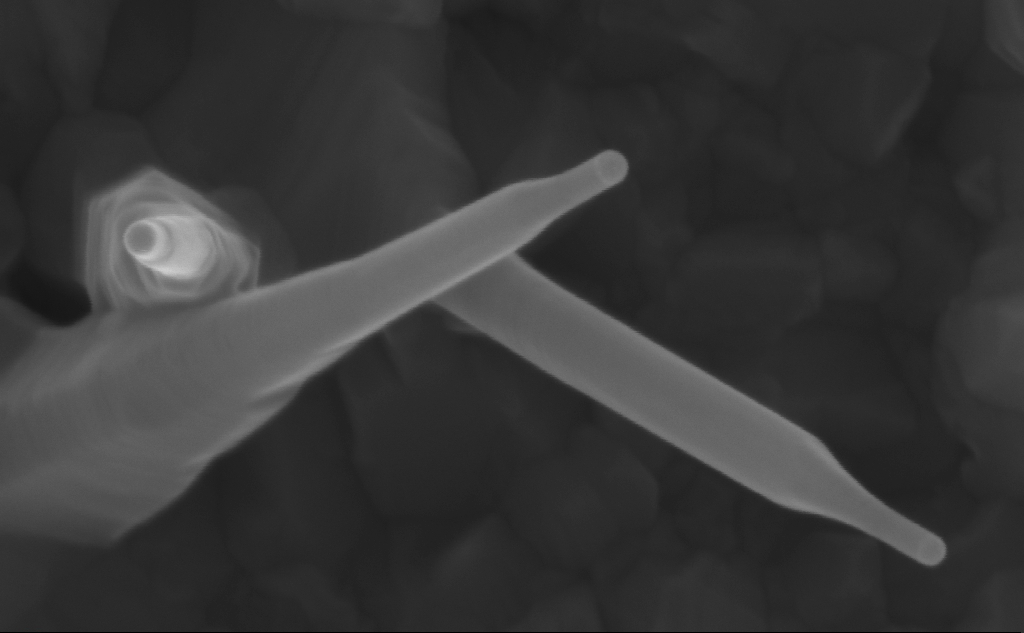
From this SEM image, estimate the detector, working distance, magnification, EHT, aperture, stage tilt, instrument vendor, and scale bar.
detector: InLens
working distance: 7 mm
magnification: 315.4 K X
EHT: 10 kV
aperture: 30 µm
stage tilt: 0°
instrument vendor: Zeiss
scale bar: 100 nm